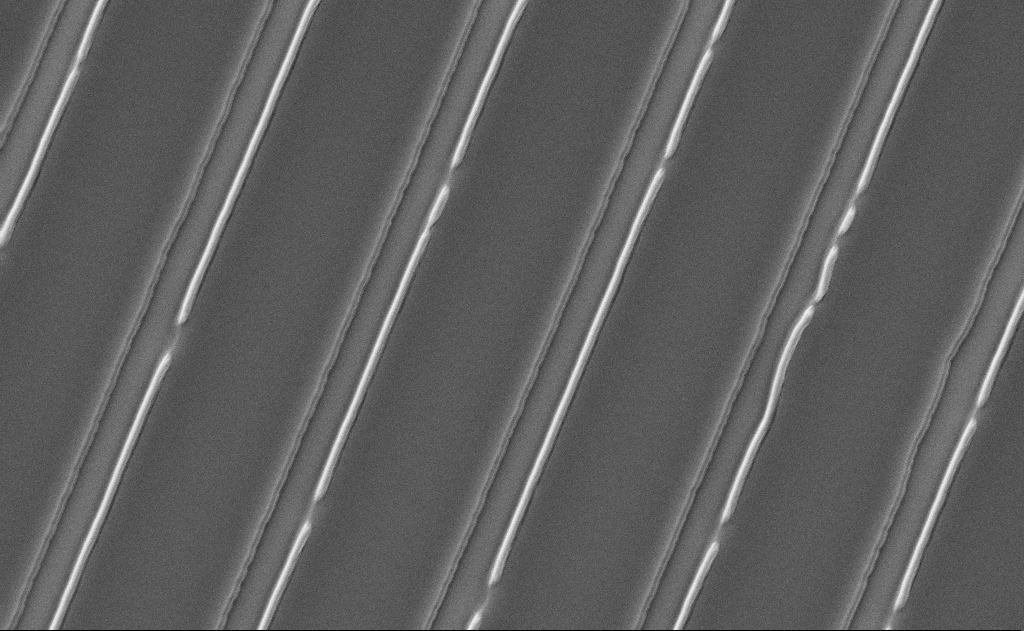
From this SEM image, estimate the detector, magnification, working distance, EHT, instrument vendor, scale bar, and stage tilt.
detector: SE2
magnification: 17.58 K X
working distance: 15 mm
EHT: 5 kV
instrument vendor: Zeiss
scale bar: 2000 nm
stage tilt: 0°